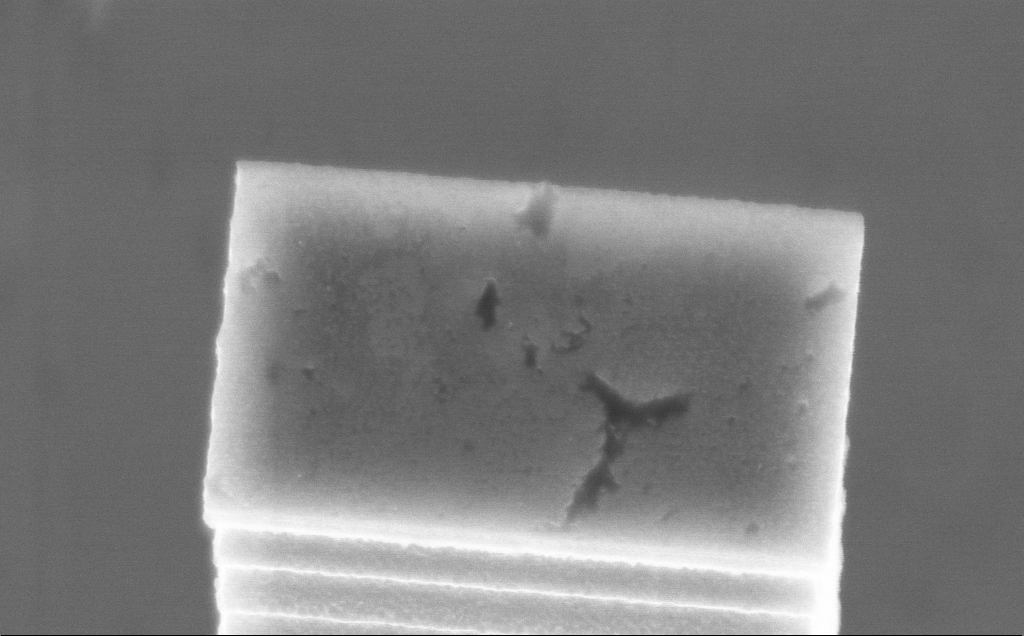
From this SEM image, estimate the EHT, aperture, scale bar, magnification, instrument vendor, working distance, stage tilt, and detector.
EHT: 7.5 kV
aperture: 30 µm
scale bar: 1000 nm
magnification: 48.05 K X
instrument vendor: Zeiss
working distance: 5 mm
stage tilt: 45°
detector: InLens